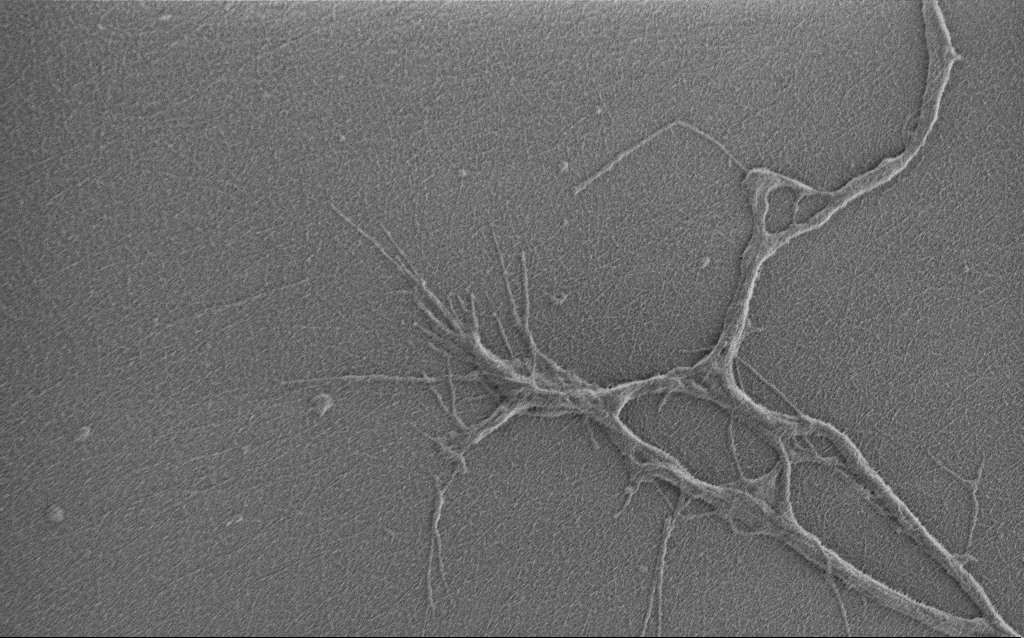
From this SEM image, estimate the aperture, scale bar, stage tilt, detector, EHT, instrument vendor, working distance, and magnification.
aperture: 30 µm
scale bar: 2000 nm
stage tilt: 0°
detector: SE2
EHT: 0.9 kV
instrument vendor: Zeiss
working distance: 6 mm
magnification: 7.5 K X